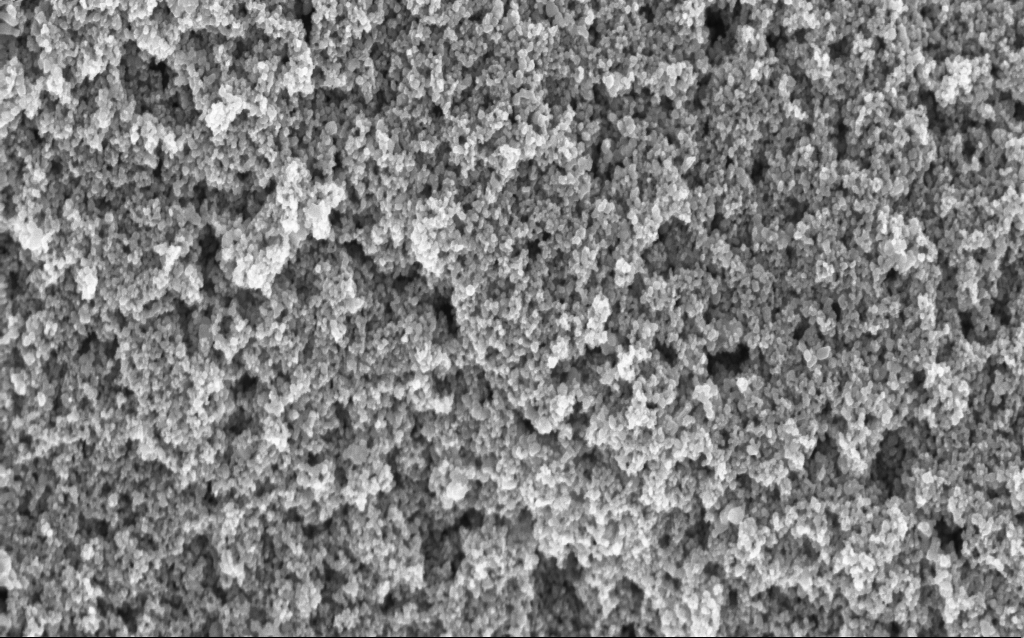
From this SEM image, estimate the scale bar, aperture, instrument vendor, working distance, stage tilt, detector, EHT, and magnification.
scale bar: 1000 nm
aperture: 30 µm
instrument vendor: Zeiss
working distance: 4.7 mm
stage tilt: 0°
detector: InLens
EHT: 5 kV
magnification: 60.3 K X